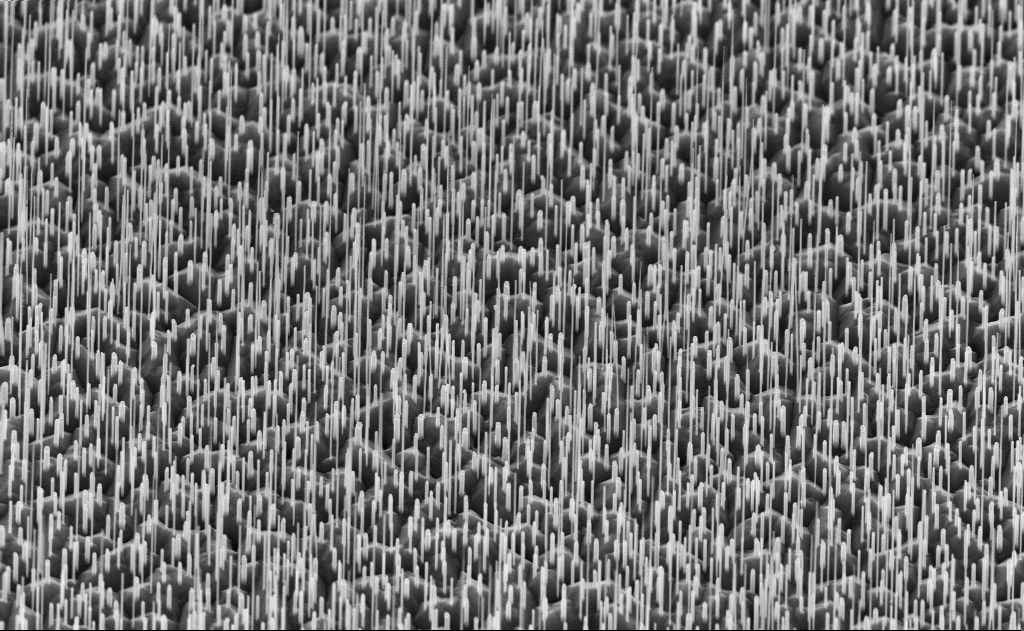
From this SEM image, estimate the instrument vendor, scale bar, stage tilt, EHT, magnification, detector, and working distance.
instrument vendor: Zeiss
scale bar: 2000 nm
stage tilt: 45°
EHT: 10 kV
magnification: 20 K X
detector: InLens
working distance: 6 mm